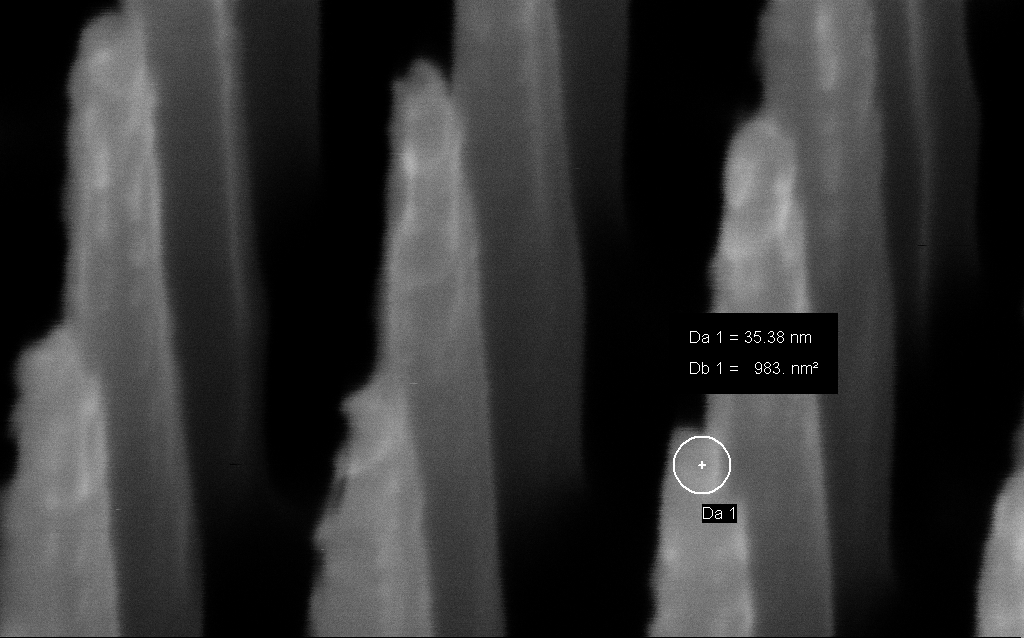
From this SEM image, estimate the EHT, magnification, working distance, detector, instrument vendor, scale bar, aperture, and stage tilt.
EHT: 3 kV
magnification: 601.93 K X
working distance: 5 mm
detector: InLens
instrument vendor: Zeiss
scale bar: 100 nm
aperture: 30 µm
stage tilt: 45°